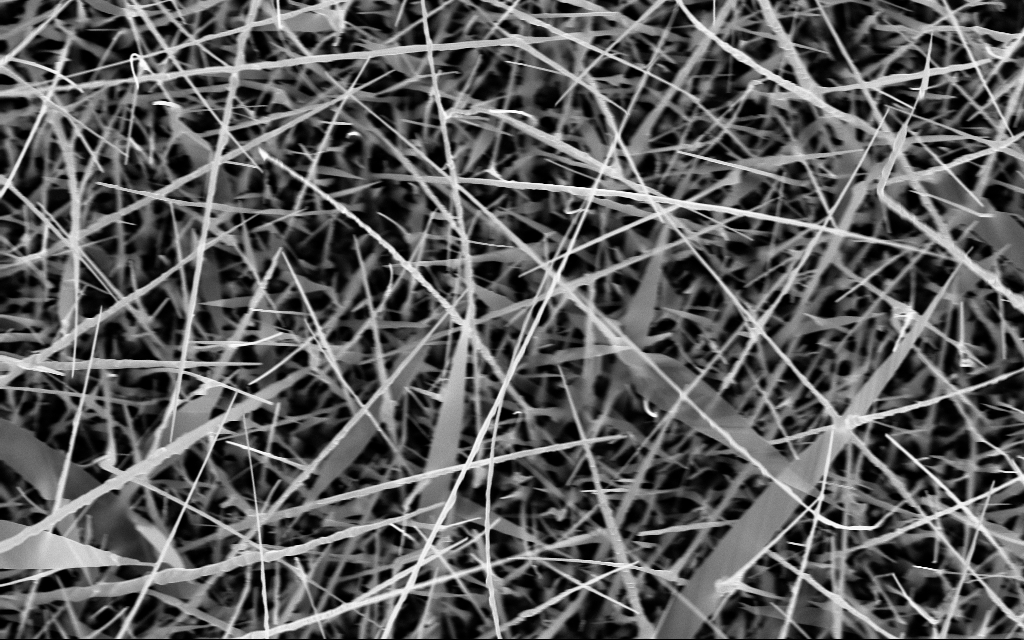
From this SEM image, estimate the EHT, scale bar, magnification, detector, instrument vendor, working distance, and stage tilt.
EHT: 10 kV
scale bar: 2000 nm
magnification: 20 K X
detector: InLens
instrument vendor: Zeiss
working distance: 6 mm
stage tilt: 0°